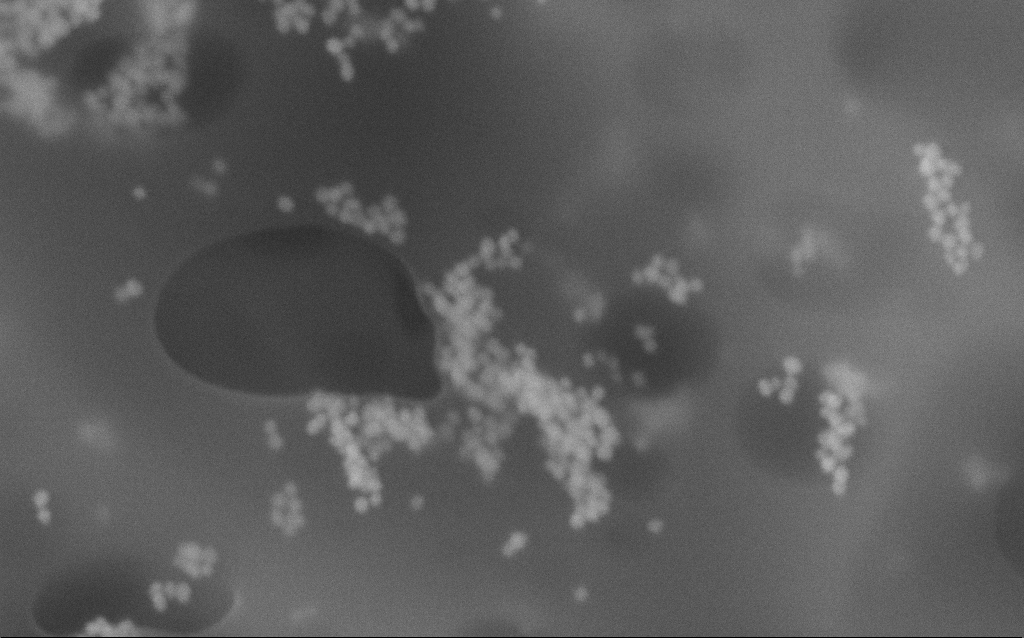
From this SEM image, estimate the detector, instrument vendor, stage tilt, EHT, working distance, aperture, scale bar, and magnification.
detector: InLens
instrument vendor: Zeiss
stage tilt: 0°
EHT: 10 kV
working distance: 3.2 mm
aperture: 30 µm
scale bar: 100 nm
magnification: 383.75 K X